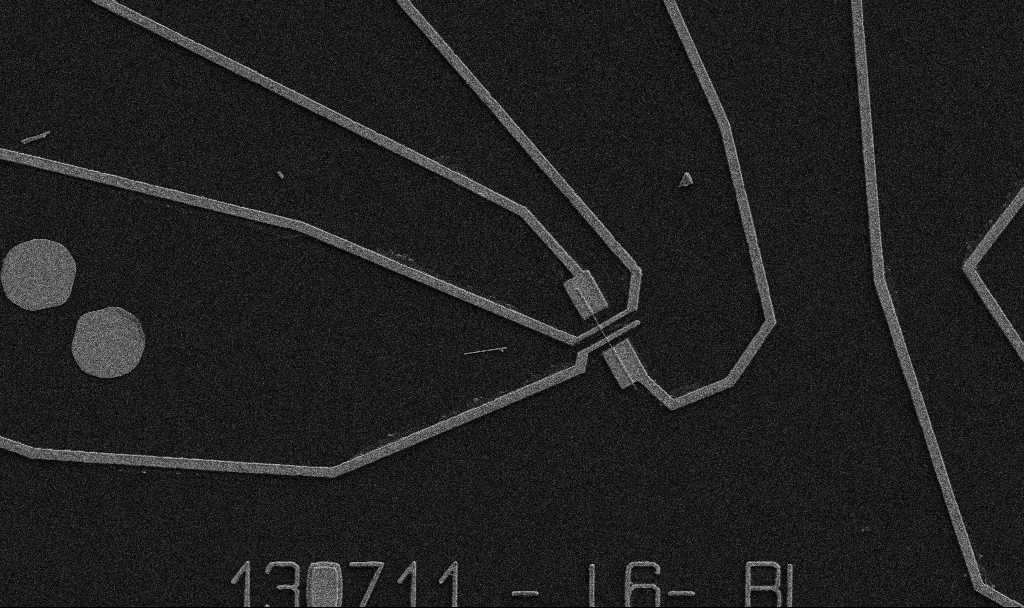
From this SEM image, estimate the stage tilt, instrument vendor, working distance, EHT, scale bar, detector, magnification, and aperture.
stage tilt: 0°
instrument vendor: Zeiss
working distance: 10.7 mm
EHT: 5 kV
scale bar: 10000 nm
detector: SE2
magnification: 5 K X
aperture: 30 µm